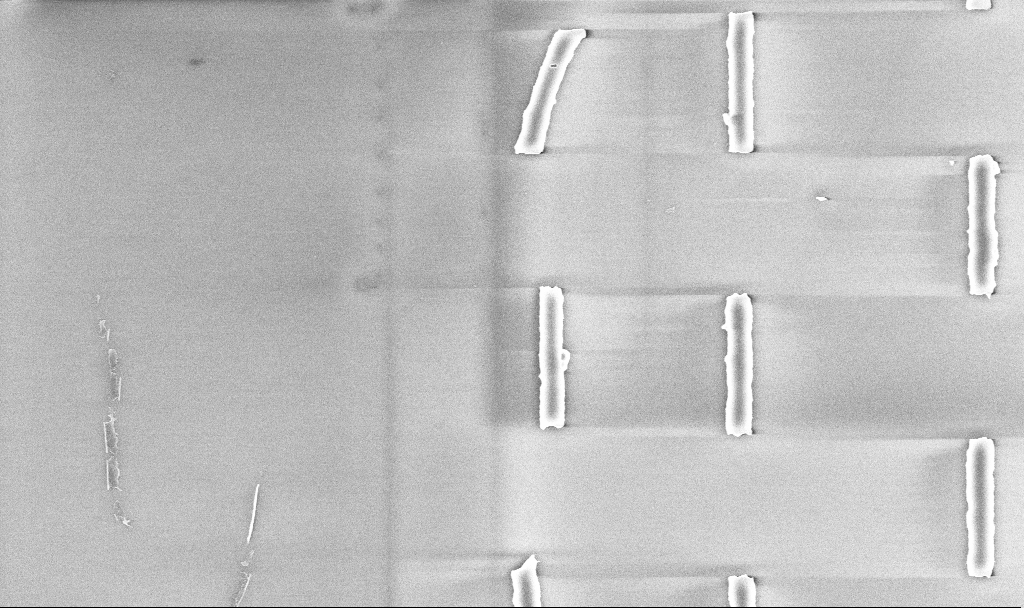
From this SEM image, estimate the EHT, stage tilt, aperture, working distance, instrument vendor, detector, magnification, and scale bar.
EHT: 5 kV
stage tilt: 0°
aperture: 30 µm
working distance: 5.2 mm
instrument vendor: Zeiss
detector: InLens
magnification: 17.44 K X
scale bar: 2000 nm